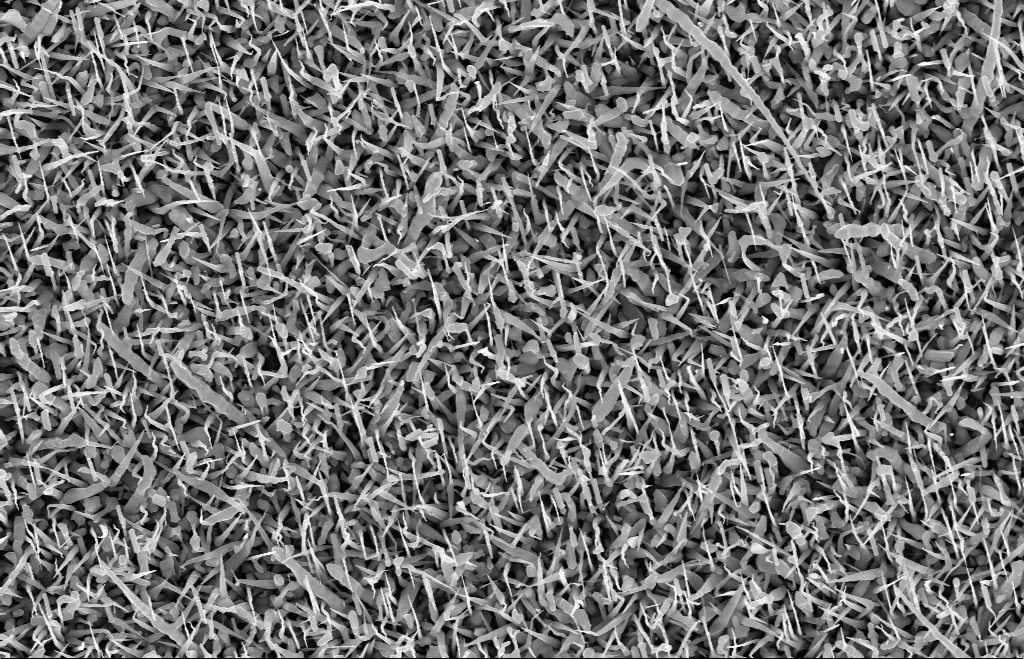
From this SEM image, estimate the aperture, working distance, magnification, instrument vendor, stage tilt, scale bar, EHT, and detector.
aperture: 30 µm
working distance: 8 mm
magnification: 20 K X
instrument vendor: Zeiss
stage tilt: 0°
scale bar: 1000 nm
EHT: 10 kV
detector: InLens